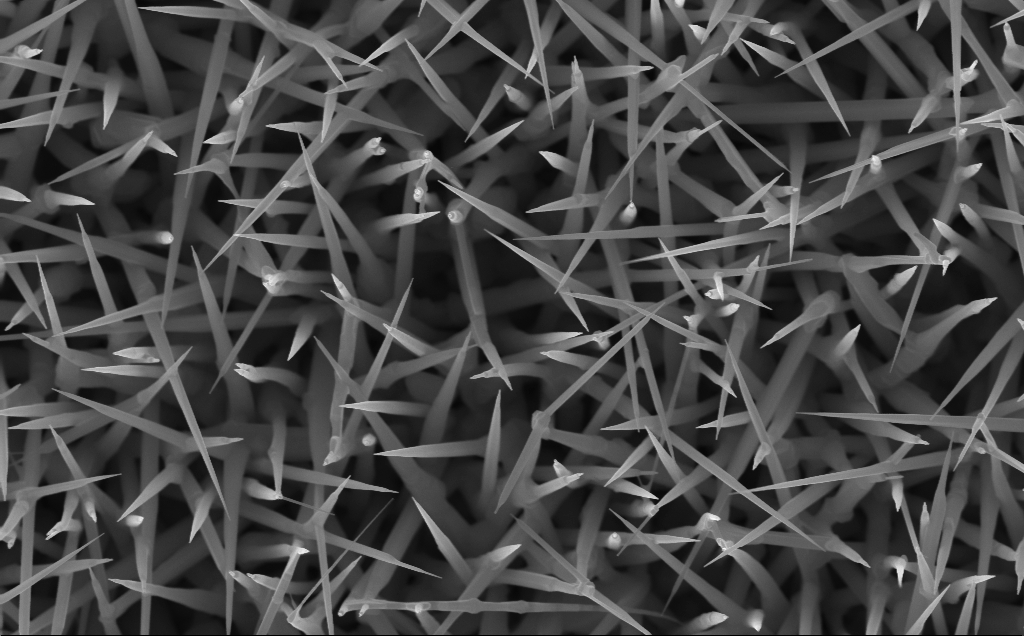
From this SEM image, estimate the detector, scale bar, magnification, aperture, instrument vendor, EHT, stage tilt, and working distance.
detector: InLens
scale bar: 1000 nm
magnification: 40 K X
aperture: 30 µm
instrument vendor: Zeiss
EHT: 10 kV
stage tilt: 0°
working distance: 5 mm